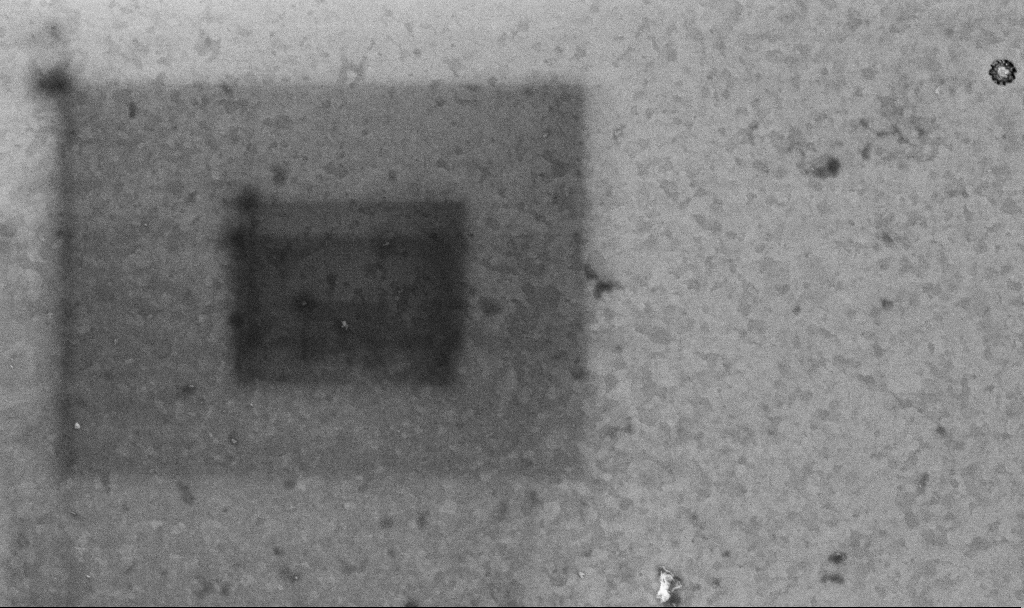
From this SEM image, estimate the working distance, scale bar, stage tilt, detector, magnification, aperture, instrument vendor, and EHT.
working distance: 3.4 mm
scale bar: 1000 nm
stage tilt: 0°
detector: InLens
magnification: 50.94 K X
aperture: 30 µm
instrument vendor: Zeiss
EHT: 10 kV